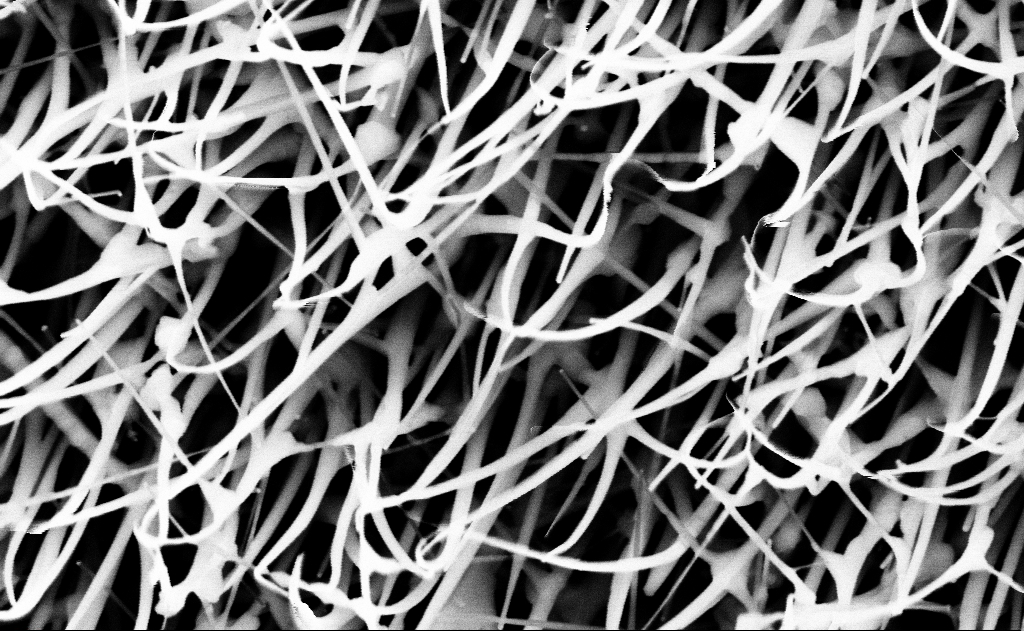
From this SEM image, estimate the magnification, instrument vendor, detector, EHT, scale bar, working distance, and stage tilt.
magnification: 80 K X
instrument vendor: Zeiss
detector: InLens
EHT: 10 kV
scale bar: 200 nm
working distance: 12 mm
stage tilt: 0°